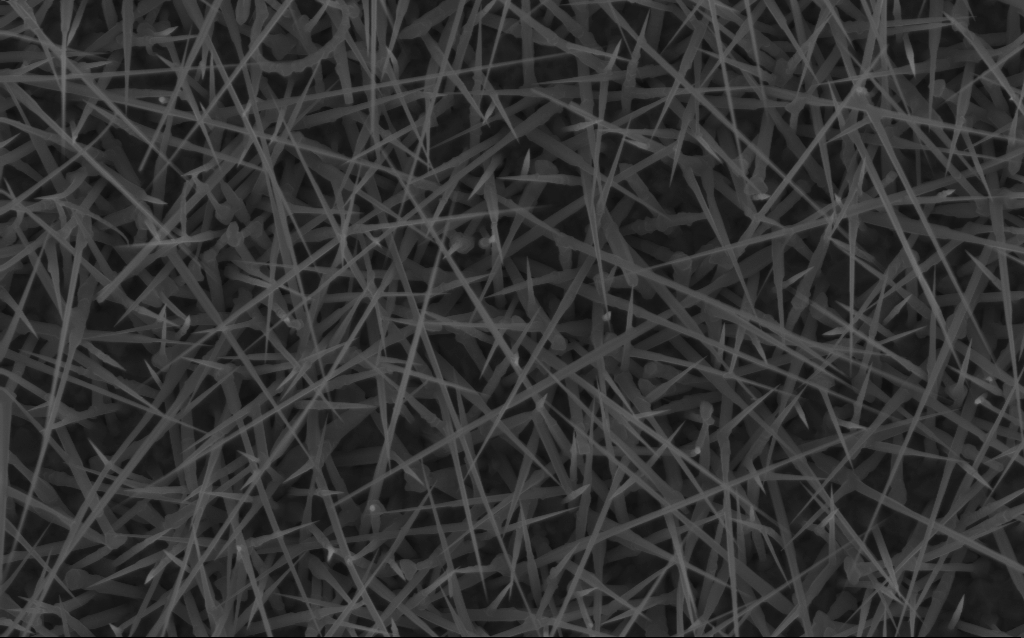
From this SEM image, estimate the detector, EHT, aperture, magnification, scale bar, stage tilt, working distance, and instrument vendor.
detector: InLens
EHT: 10 kV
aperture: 30 µm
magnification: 20 K X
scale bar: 2000 nm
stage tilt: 0°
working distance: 7 mm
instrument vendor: Zeiss